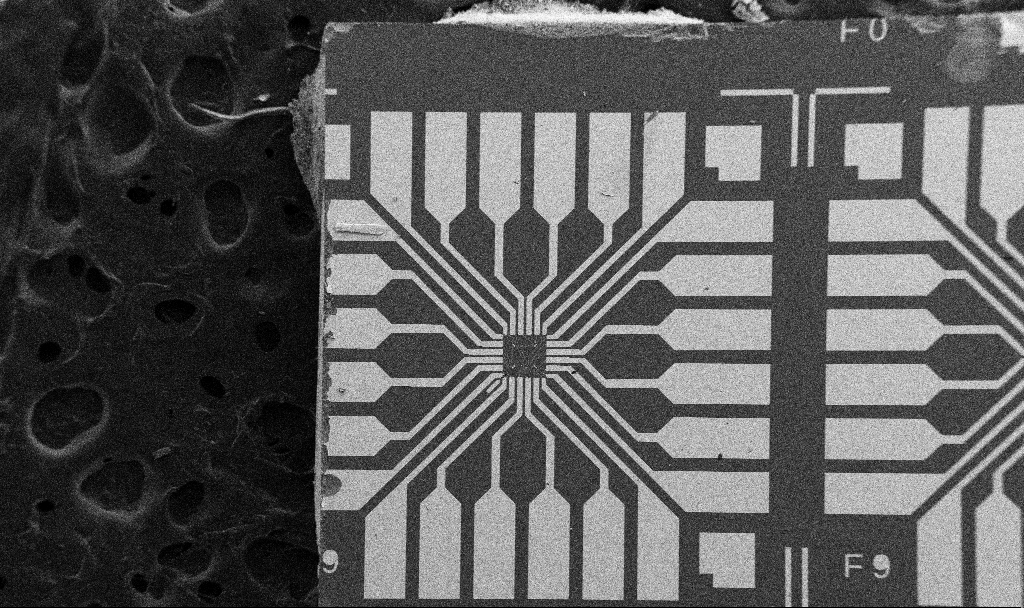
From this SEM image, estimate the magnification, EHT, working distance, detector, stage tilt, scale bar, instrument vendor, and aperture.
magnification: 0.1 K X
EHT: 5 kV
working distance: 10.7 mm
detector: SE2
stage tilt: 0°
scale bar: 200000 nm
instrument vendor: Zeiss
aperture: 30 µm